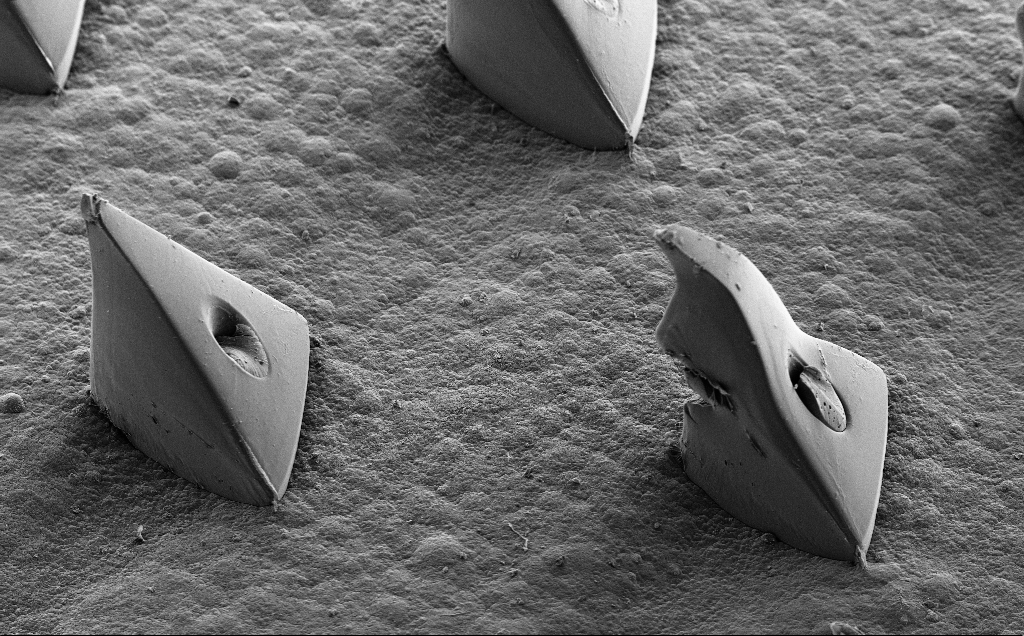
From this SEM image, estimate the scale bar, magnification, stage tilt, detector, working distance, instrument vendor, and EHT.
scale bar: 200000 nm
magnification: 0.144 K X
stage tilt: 35.3°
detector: SE2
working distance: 10 mm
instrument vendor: Zeiss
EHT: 10 kV